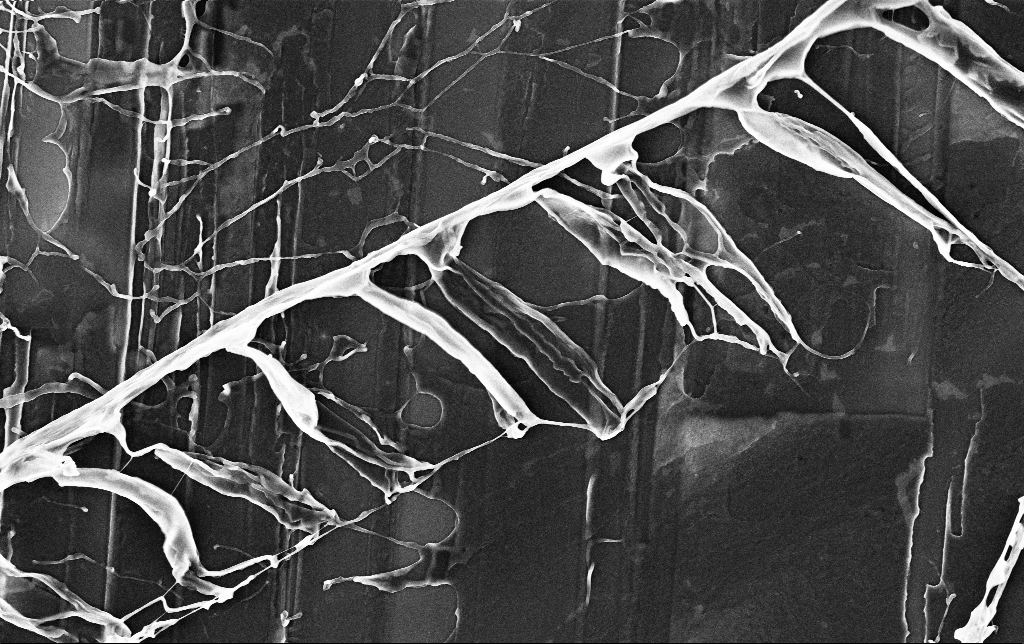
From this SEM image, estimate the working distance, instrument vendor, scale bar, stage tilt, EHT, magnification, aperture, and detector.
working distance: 3.5 mm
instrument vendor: Zeiss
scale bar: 2000 nm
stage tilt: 0°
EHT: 3 kV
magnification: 13.05 K X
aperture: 30 µm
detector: InLens